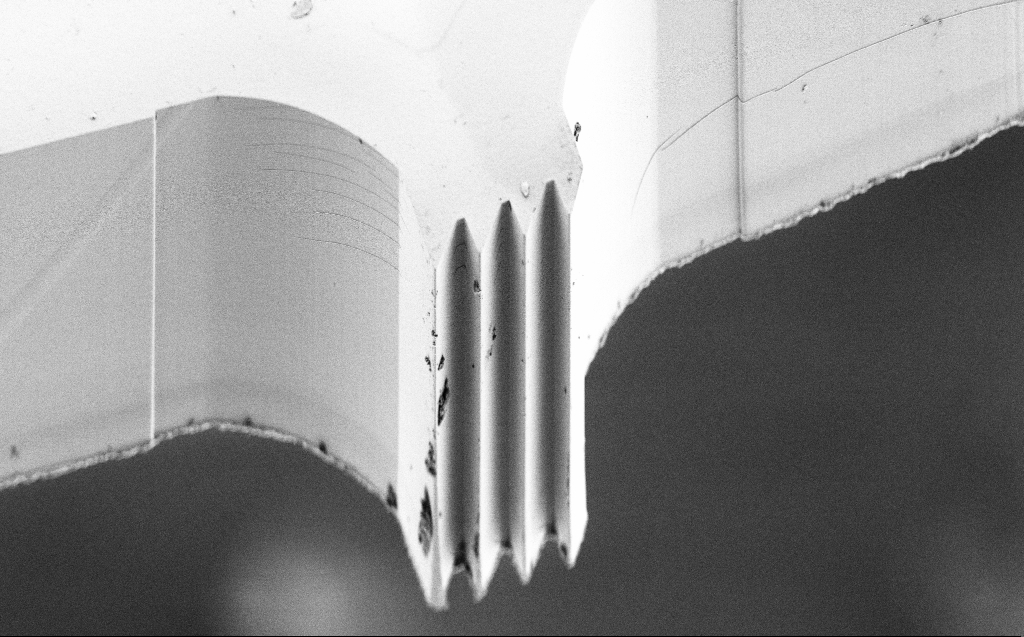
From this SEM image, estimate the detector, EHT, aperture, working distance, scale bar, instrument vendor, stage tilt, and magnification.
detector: SE2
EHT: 3 kV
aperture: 30 µm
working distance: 8 mm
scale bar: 10000 nm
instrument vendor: Zeiss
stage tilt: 45°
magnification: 1.54 K X